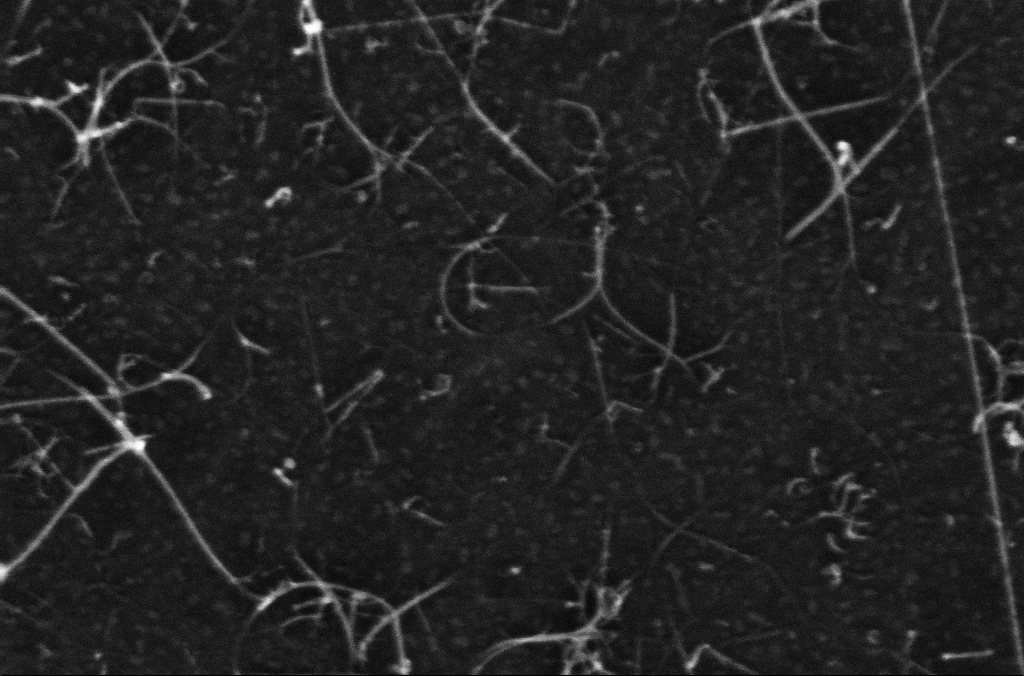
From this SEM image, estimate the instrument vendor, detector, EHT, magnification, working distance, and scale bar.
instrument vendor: Zeiss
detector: InLens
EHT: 10 kV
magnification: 272.1 K X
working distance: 3.3 mm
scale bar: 200 nm